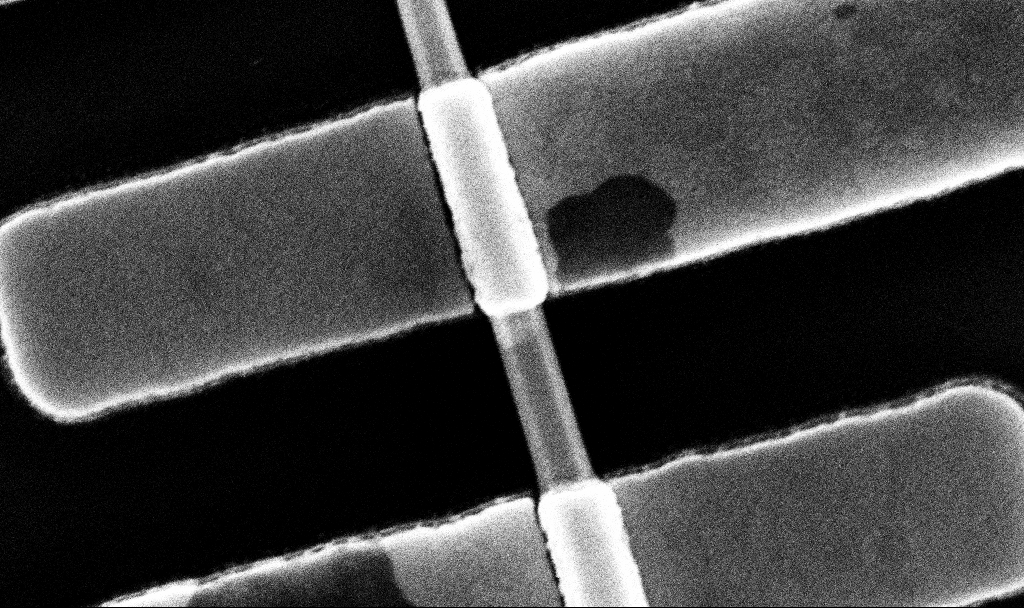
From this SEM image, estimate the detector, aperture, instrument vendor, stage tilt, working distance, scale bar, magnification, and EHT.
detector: InLens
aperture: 30 µm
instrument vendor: Zeiss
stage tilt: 0°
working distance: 6.7 mm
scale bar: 200 nm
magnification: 136.1 K X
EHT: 10 kV